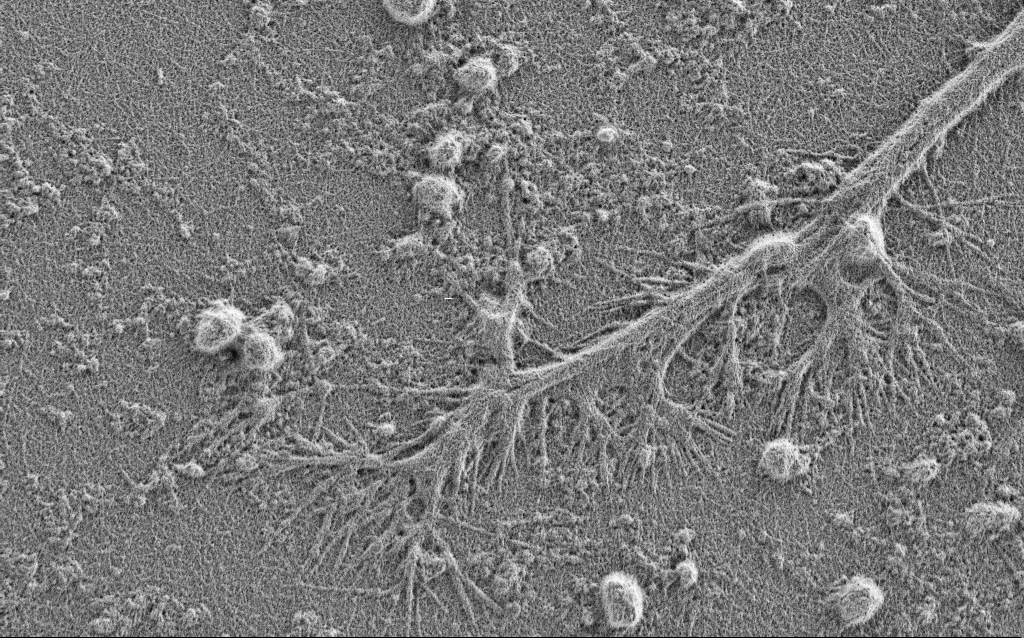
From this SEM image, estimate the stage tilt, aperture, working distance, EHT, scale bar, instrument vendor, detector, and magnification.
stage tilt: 0°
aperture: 30 µm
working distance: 4 mm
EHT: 0.9 kV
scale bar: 2000 nm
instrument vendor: Zeiss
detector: SE2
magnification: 7.5 K X